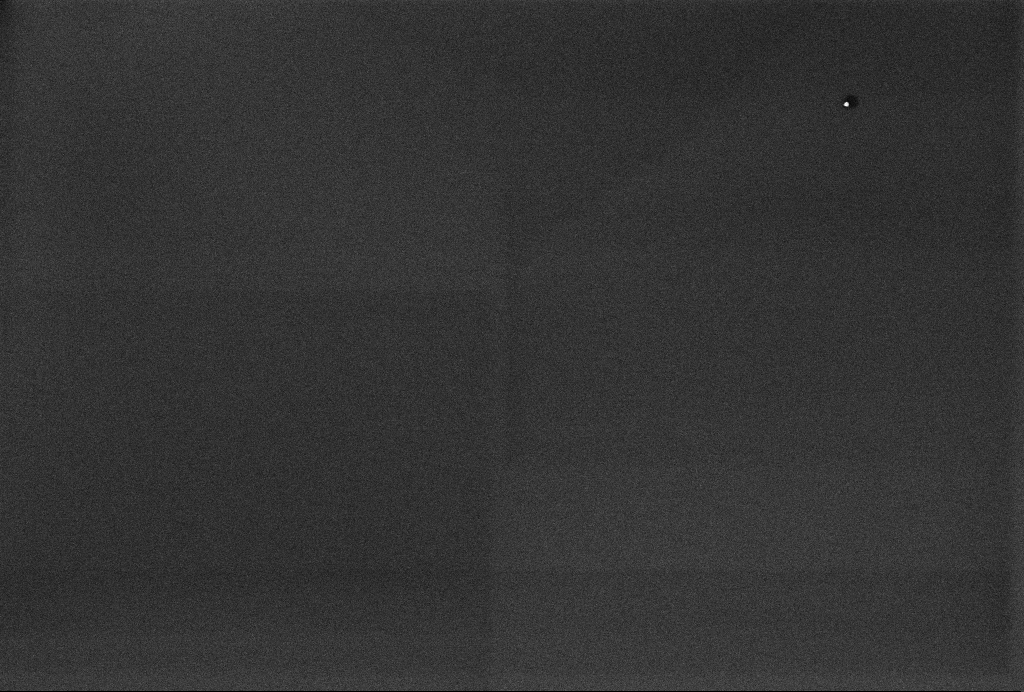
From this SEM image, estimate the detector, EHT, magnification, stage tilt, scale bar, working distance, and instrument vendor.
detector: InLens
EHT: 2 kV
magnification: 60 K X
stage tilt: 0°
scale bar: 1000 nm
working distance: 3.3 mm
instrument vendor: Zeiss